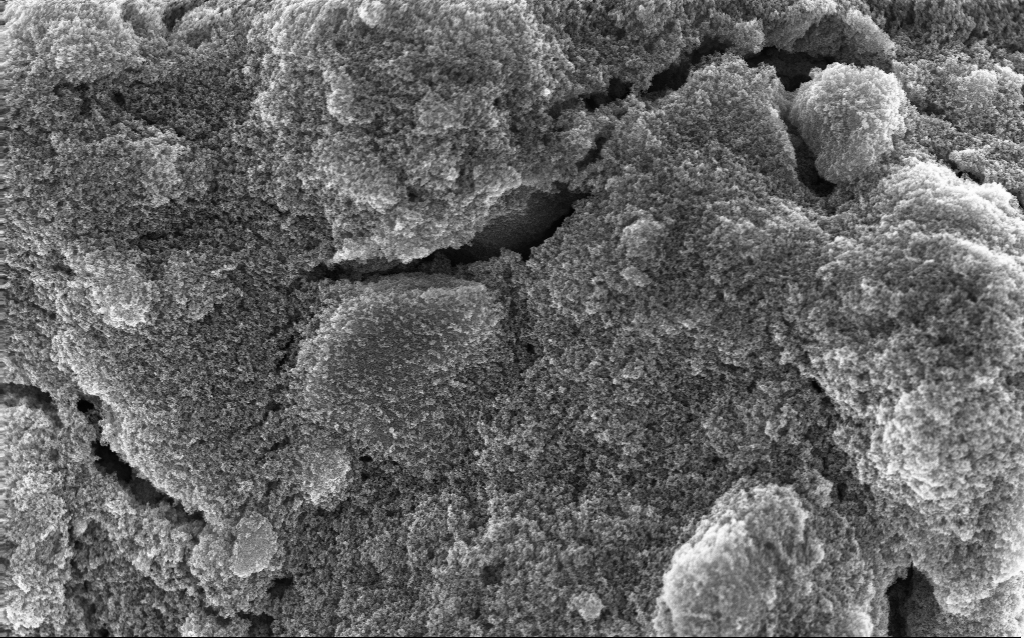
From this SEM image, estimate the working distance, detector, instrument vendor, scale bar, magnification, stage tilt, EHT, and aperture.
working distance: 2.6 mm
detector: InLens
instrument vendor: Zeiss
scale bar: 1000 nm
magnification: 20.87 K X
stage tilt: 0°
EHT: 10 kV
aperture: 30 µm